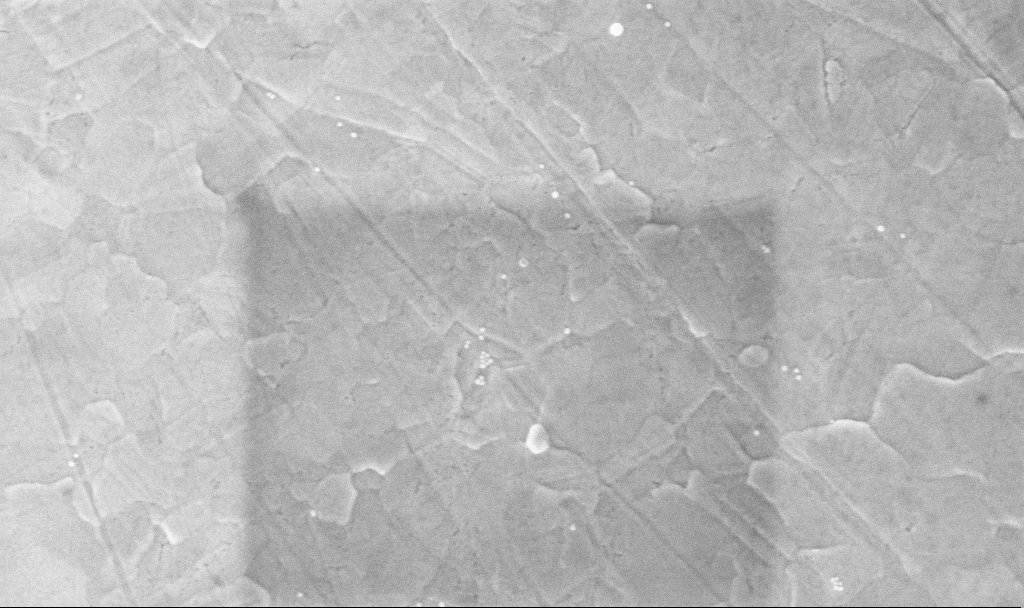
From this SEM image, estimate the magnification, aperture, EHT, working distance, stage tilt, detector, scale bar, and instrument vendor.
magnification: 100 K X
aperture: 30 µm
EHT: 5 kV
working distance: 3.7 mm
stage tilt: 0°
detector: InLens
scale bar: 200 nm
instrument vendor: Zeiss